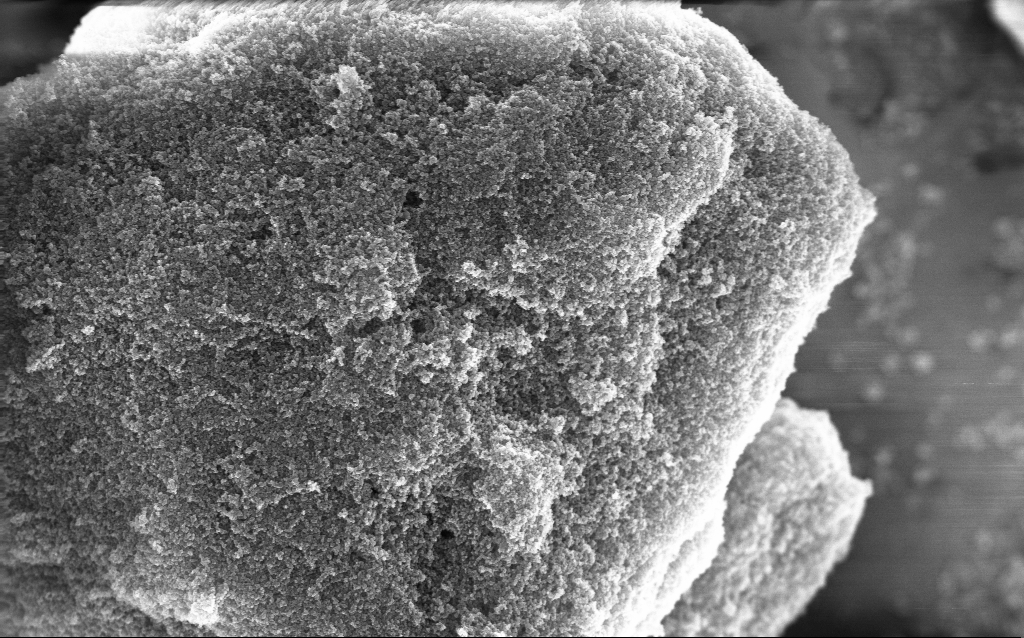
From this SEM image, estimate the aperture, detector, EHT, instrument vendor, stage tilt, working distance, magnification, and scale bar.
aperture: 30 µm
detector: InLens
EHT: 10 kV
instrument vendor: Zeiss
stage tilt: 0°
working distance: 2.8 mm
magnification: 37.88 K X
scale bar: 1000 nm